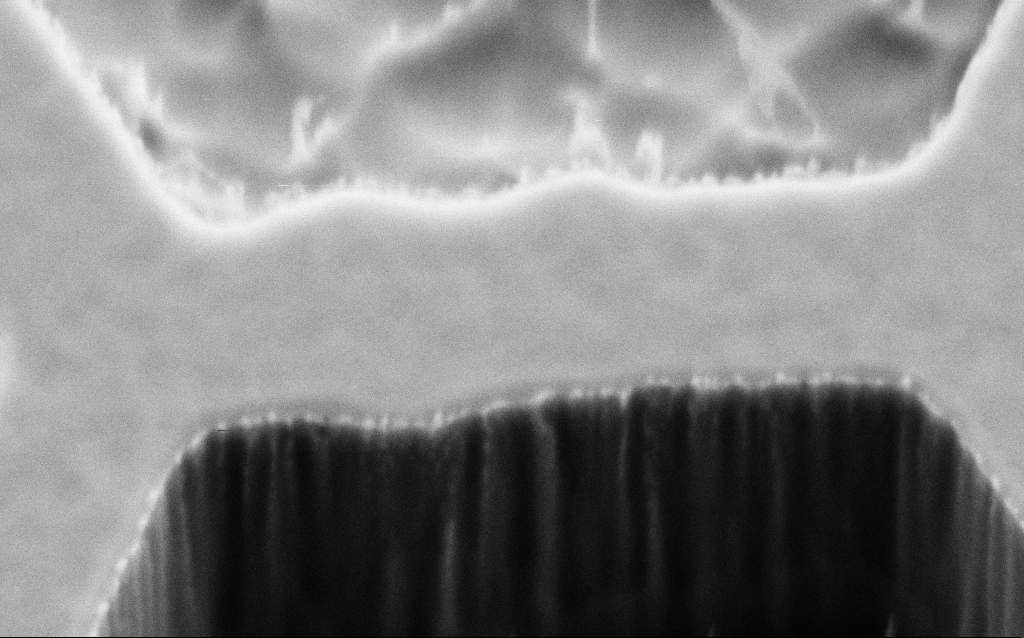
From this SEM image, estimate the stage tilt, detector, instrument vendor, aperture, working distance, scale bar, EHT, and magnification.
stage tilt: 45°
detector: SE2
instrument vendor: Zeiss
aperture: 30 µm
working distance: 6 mm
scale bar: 200 nm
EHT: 2 kV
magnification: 176.18 K X